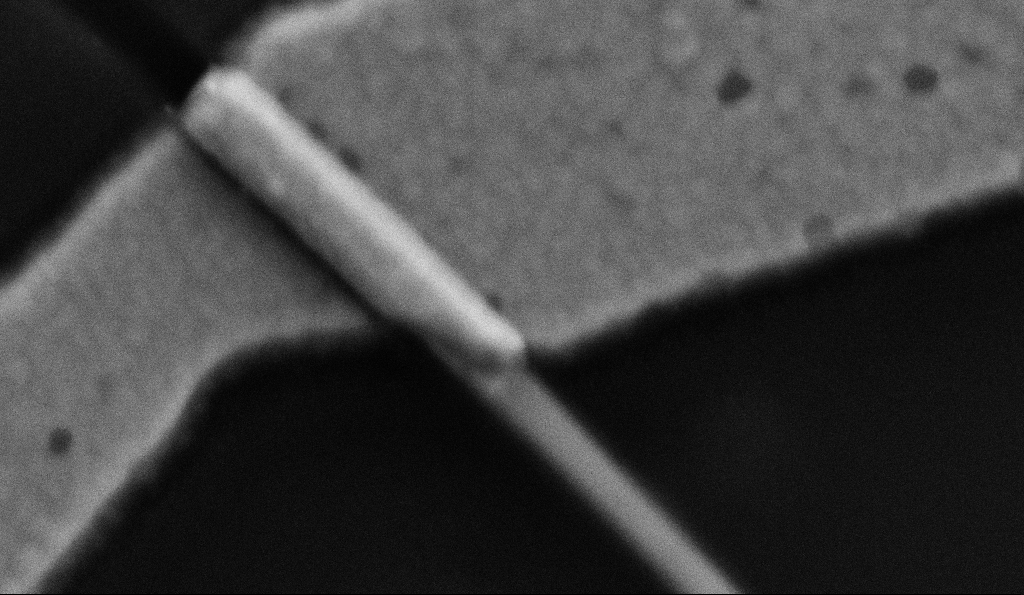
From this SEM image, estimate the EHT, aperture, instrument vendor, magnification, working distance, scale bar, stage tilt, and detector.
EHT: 5 kV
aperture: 30 µm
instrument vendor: Zeiss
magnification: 200 K X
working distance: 8.5 mm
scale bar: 200 nm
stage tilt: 0°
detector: SE2